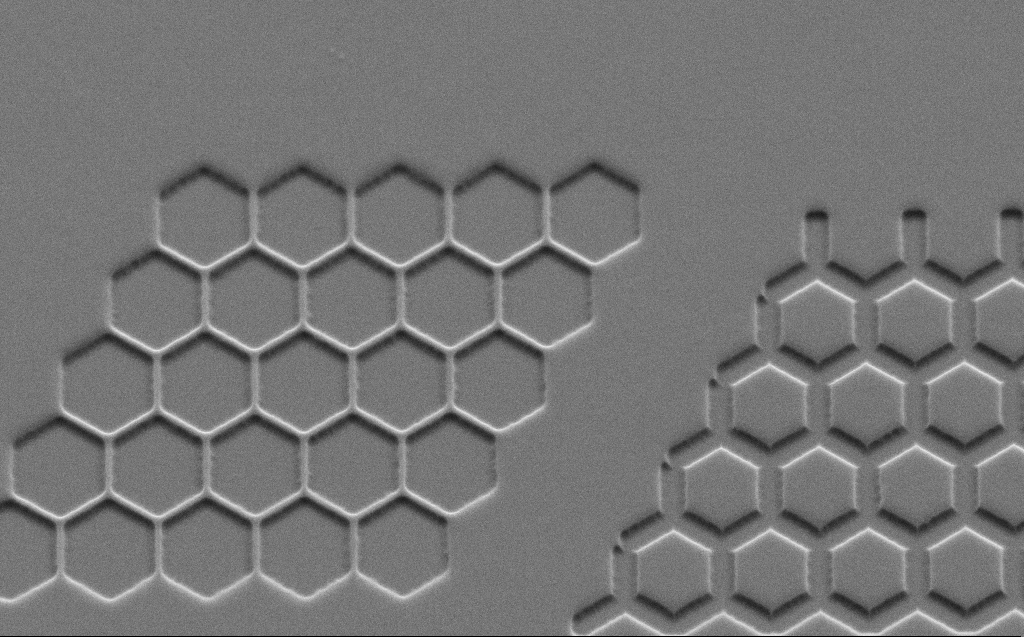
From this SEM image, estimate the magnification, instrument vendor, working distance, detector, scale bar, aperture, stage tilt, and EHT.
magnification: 5.81 K X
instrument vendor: Zeiss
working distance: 6 mm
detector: SE2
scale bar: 10000 nm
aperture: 30 µm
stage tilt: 45°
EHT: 5 kV